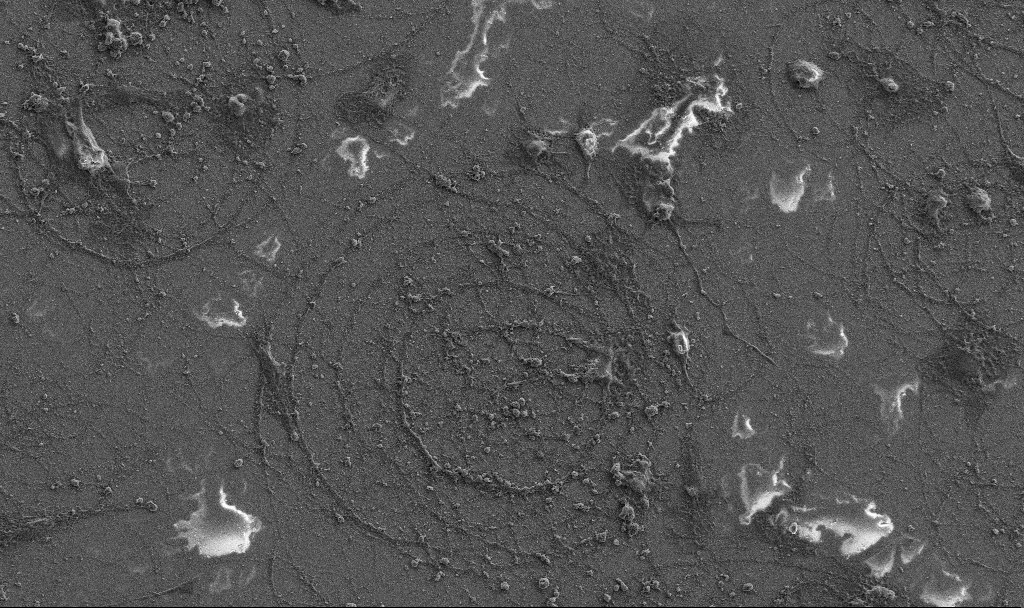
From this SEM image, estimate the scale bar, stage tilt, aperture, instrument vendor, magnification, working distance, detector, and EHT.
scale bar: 20000 nm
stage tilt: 0°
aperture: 30 µm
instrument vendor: Zeiss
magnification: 1 K X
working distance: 6.8 mm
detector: SE2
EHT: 5 kV